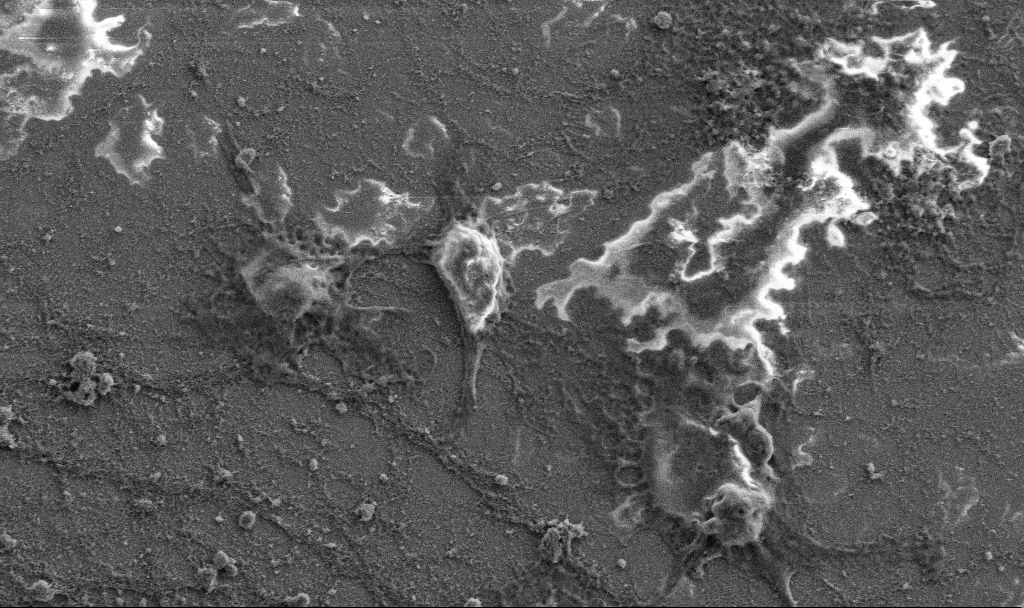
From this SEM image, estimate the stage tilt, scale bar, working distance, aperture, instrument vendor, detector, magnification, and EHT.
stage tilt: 0°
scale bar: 20000 nm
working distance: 6.8 mm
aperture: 30 µm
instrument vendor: Zeiss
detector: SE2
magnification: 3.5 K X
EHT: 5 kV